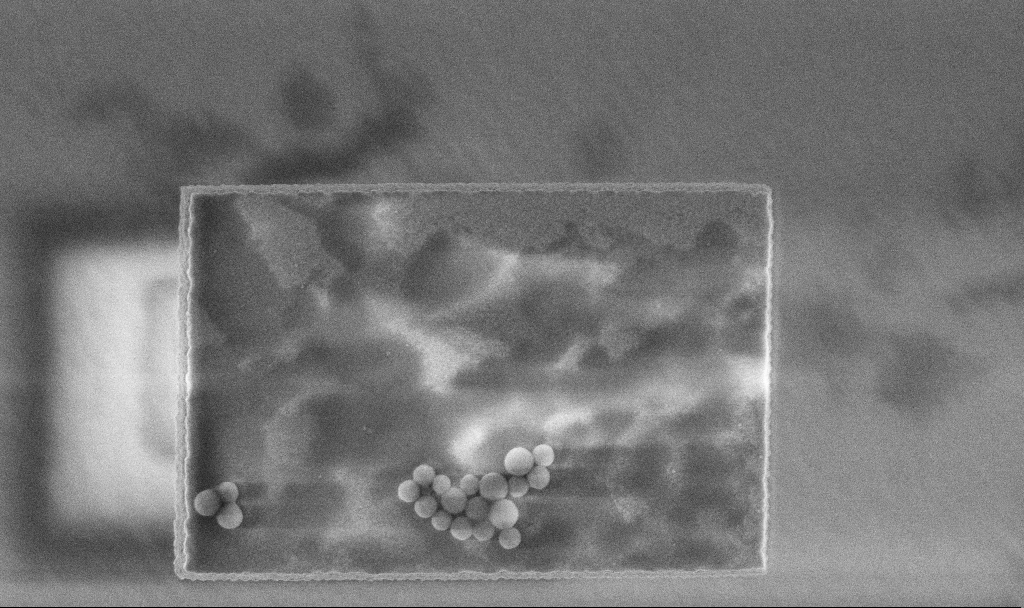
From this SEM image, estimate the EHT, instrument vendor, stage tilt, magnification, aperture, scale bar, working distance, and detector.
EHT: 3 kV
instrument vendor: Zeiss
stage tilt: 0°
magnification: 47.93 K X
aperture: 30 µm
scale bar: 1000 nm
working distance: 3.3 mm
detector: InLens